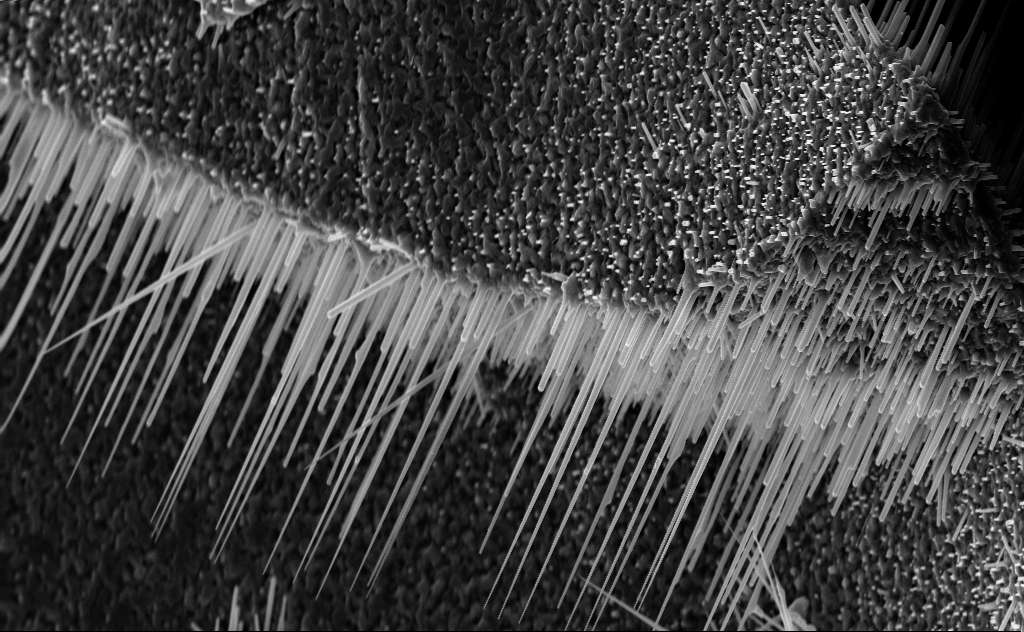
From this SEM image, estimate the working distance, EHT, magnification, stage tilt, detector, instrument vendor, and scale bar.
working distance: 8 mm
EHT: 10 kV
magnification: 10 K X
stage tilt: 0°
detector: InLens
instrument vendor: Zeiss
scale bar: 2000 nm